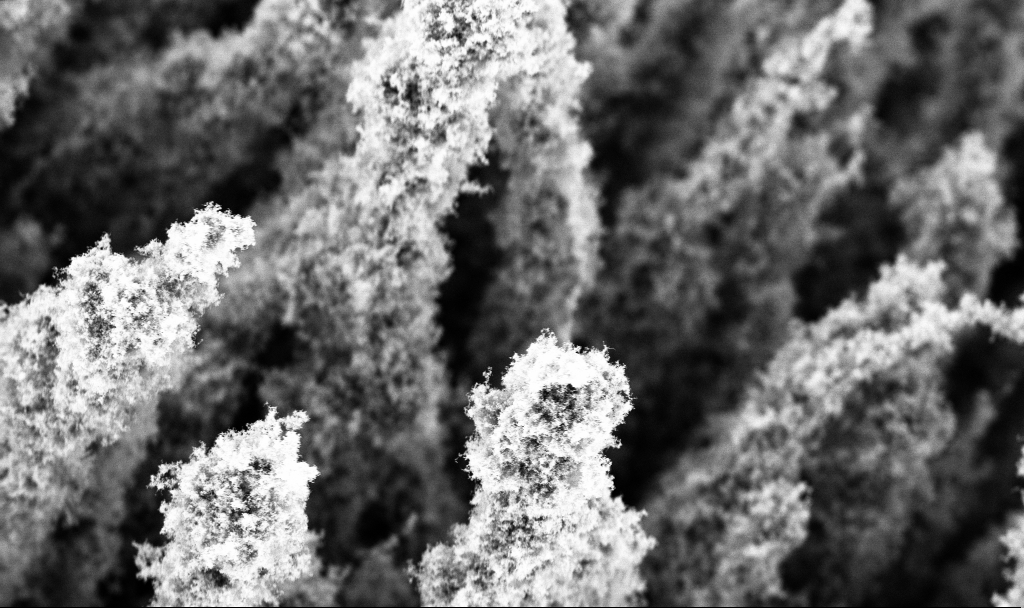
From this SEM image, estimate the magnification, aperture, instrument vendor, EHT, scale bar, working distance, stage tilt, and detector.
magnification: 5 K X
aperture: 30 µm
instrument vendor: Zeiss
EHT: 3 kV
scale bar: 10000 nm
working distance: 4 mm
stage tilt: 0°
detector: InLens